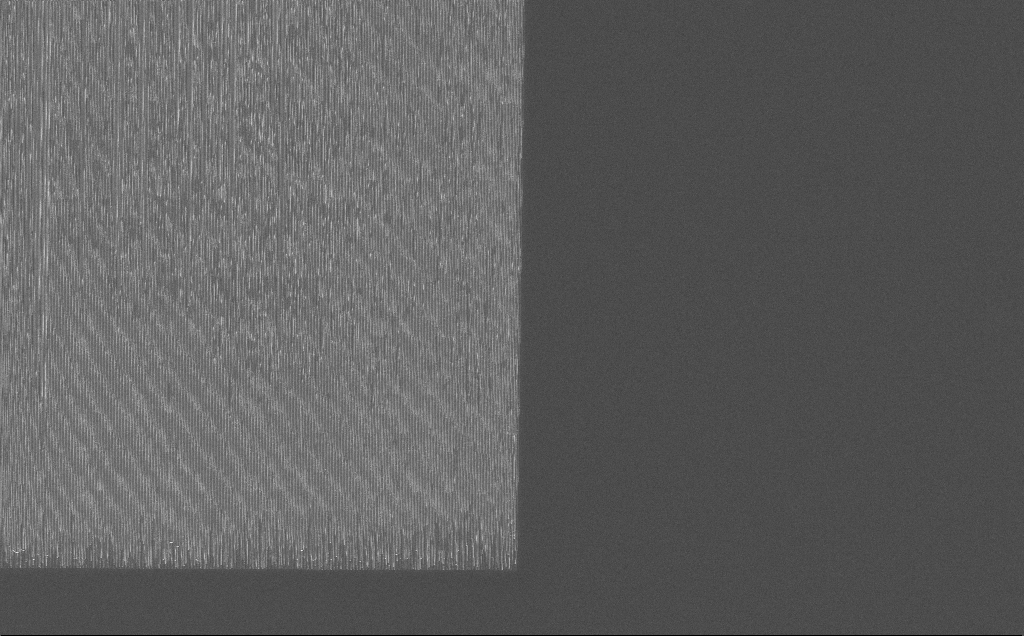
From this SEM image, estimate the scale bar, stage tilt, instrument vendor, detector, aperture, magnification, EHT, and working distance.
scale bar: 10000 nm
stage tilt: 0°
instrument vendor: Zeiss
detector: InLens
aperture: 30 µm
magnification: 2.82 K X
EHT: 10 kV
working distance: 7 mm